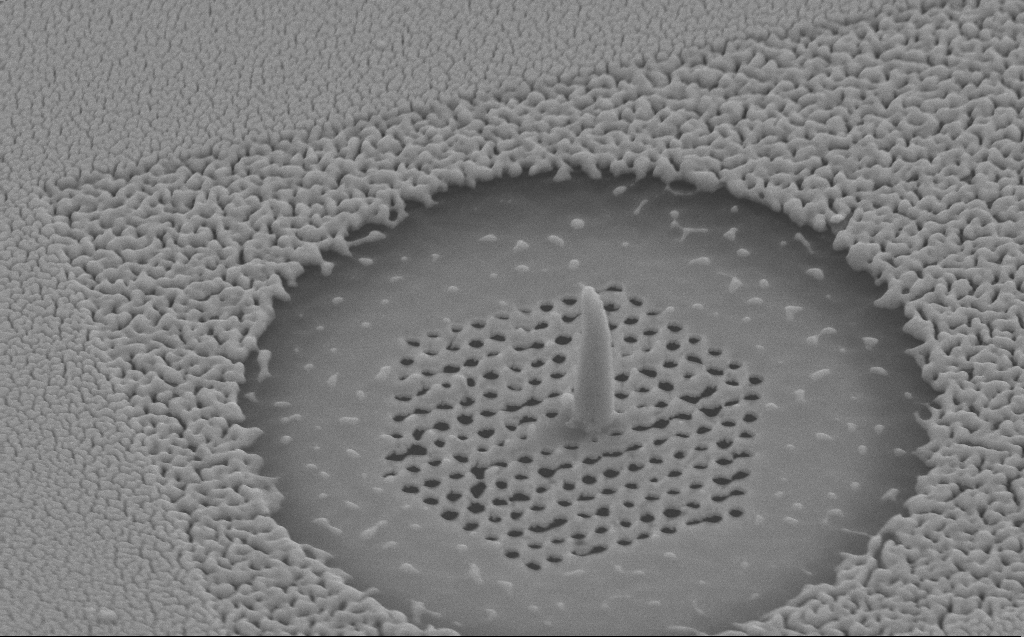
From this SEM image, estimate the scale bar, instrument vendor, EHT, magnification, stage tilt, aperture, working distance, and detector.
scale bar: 1000 nm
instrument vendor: Zeiss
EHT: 10 kV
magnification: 35.47 K X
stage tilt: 45°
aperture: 30 µm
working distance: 6 mm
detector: SE2